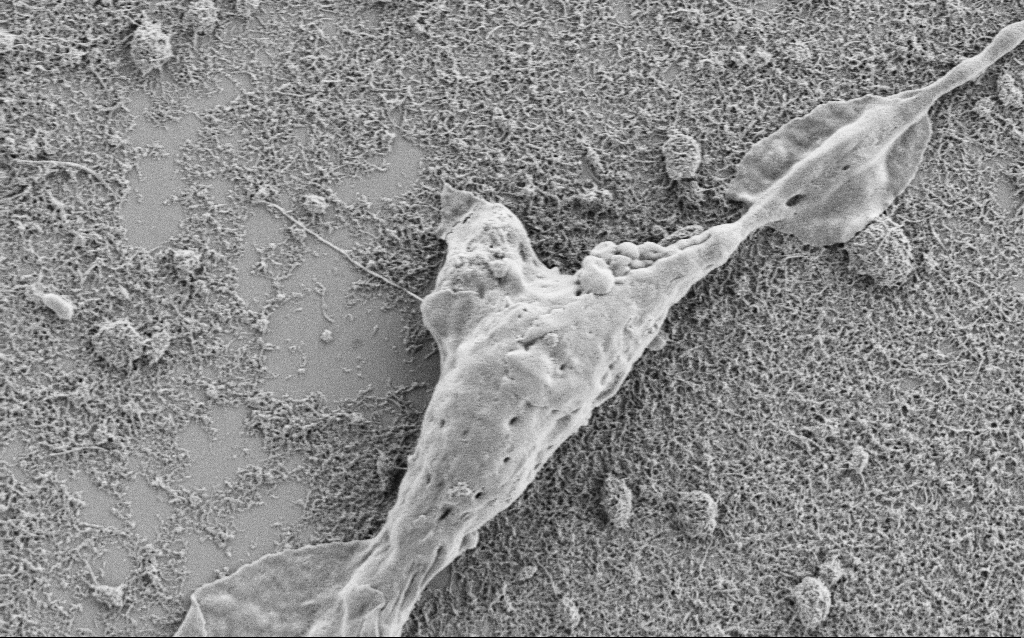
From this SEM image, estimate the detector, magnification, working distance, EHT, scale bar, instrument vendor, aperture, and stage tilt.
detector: SE2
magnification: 10 K X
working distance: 6.8 mm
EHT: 1.5 kV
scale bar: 2000 nm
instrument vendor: Zeiss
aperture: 30 µm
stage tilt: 0°